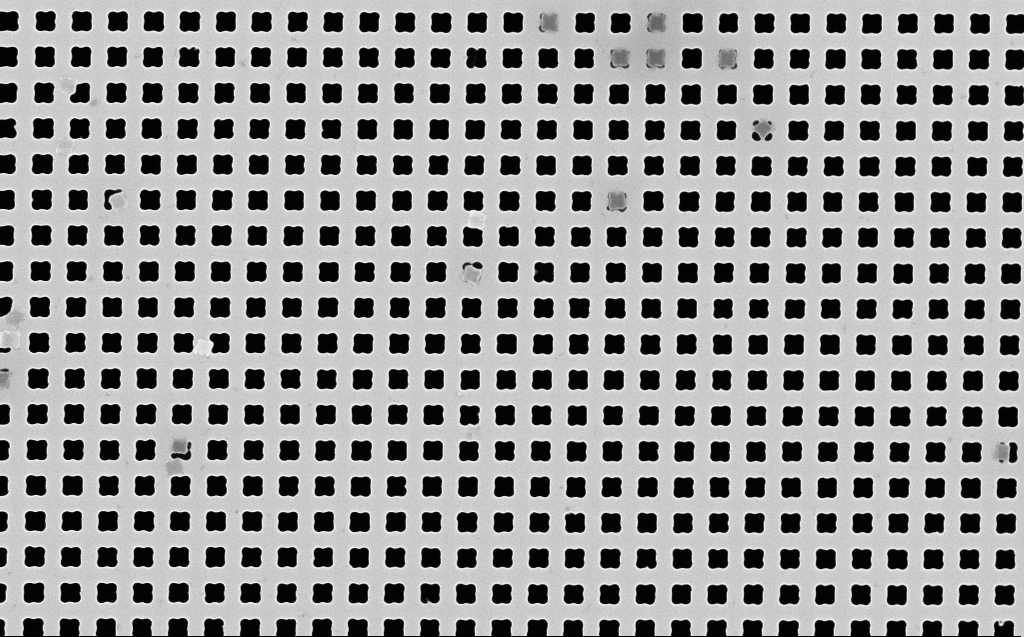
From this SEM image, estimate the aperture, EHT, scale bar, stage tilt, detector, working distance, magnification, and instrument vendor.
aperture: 30 µm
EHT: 10 kV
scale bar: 2000 nm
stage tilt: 0°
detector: InLens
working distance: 7 mm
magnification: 26.54 K X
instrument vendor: Zeiss